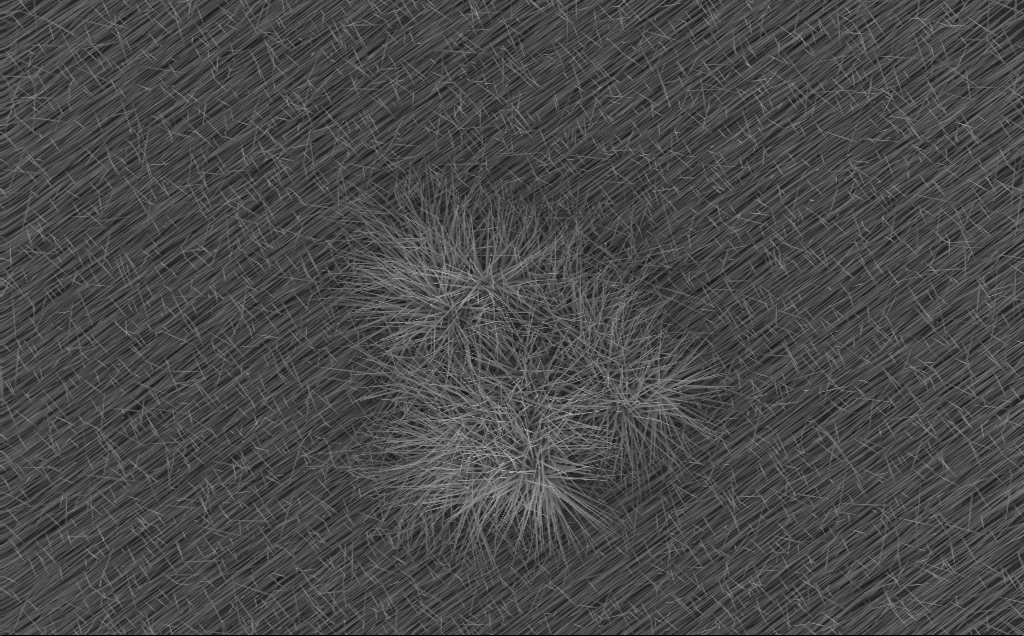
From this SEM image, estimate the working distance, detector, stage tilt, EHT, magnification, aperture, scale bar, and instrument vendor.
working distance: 6 mm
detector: InLens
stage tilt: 0°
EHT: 10 kV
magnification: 6.04 K X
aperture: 30 µm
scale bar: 10000 nm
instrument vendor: Zeiss